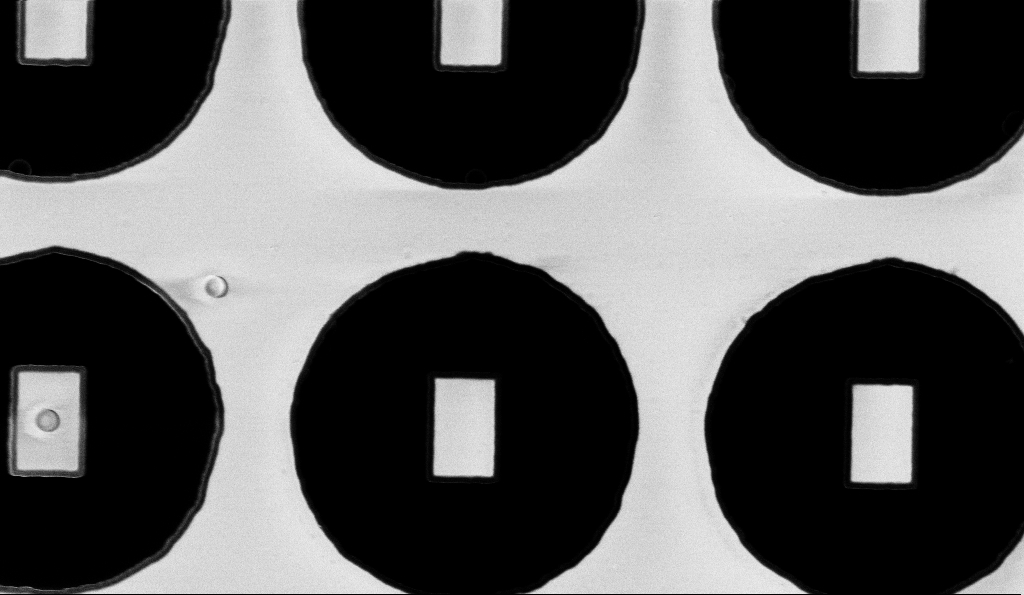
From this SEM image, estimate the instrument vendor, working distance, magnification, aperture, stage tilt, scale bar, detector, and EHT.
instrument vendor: Zeiss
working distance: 5.3 mm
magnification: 13.8 K X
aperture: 30 µm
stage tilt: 0°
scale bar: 2000 nm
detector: InLens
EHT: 3 kV